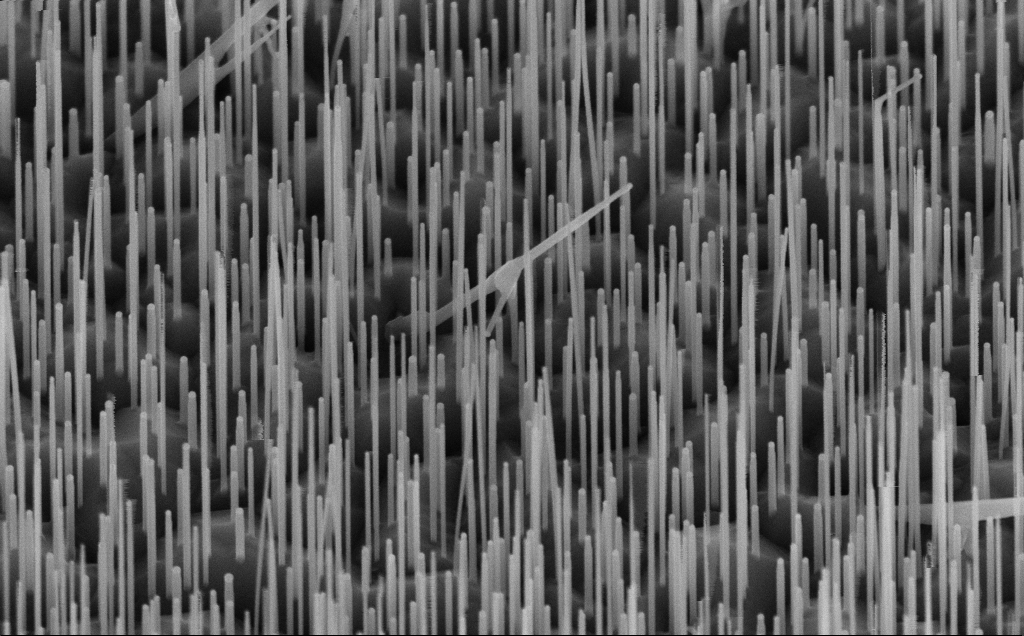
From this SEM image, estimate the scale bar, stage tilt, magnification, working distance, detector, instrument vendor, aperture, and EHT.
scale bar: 1000 nm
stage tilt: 45°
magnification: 60 K X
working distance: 7 mm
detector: InLens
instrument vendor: Zeiss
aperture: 30 µm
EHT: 10 kV